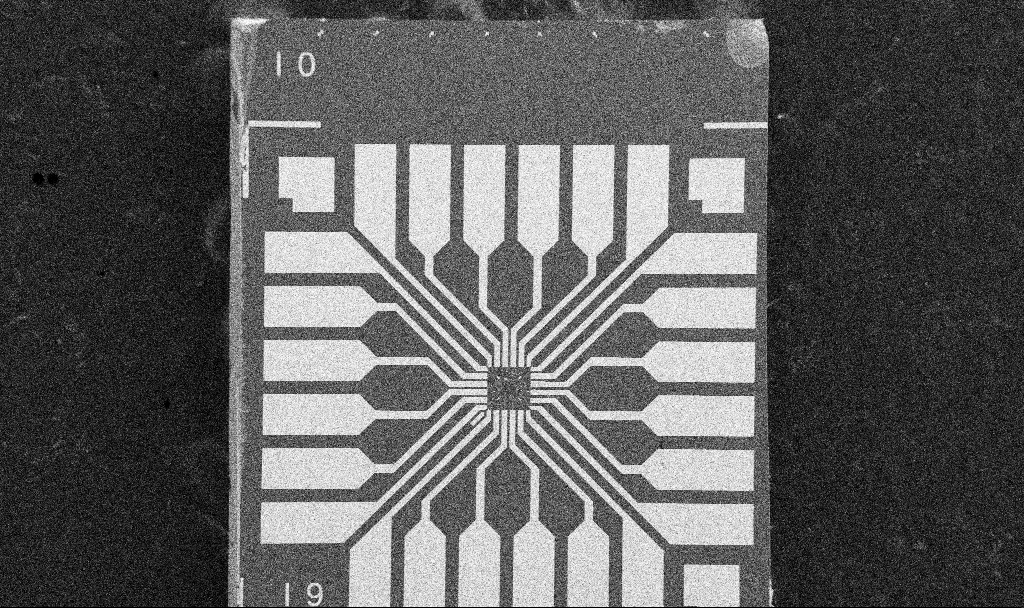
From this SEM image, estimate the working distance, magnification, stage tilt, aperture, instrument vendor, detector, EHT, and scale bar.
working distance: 10.5 mm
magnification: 0.1 K X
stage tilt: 0°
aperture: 30 µm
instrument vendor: Zeiss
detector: SE2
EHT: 5 kV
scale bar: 200000 nm